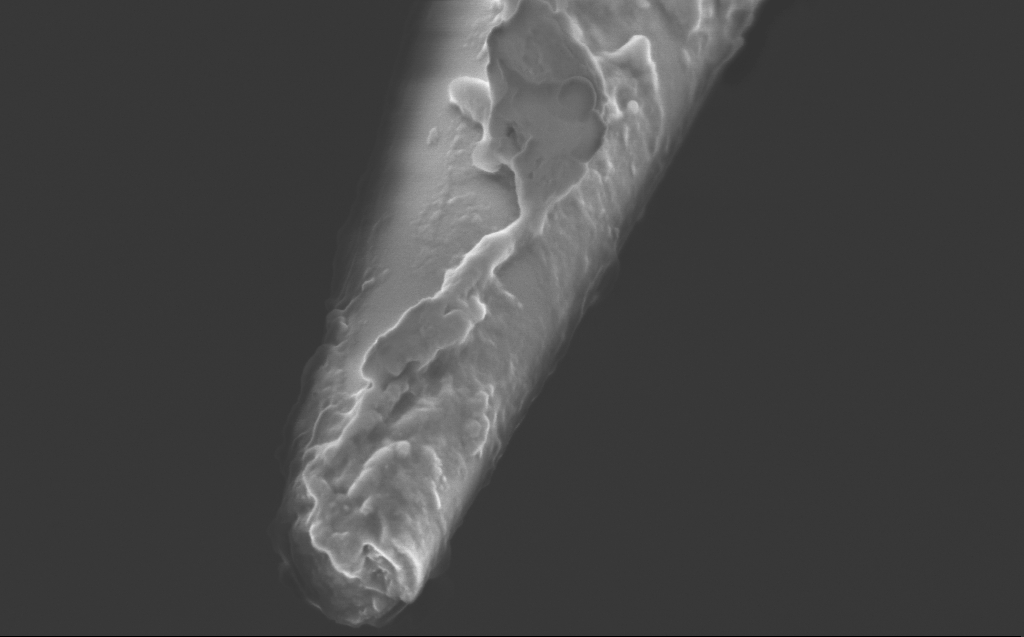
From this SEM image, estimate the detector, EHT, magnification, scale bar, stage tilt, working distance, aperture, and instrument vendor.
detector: InLens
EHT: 2 kV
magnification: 100 K X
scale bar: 200 nm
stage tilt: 45°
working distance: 3 mm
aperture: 30 µm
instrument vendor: Zeiss